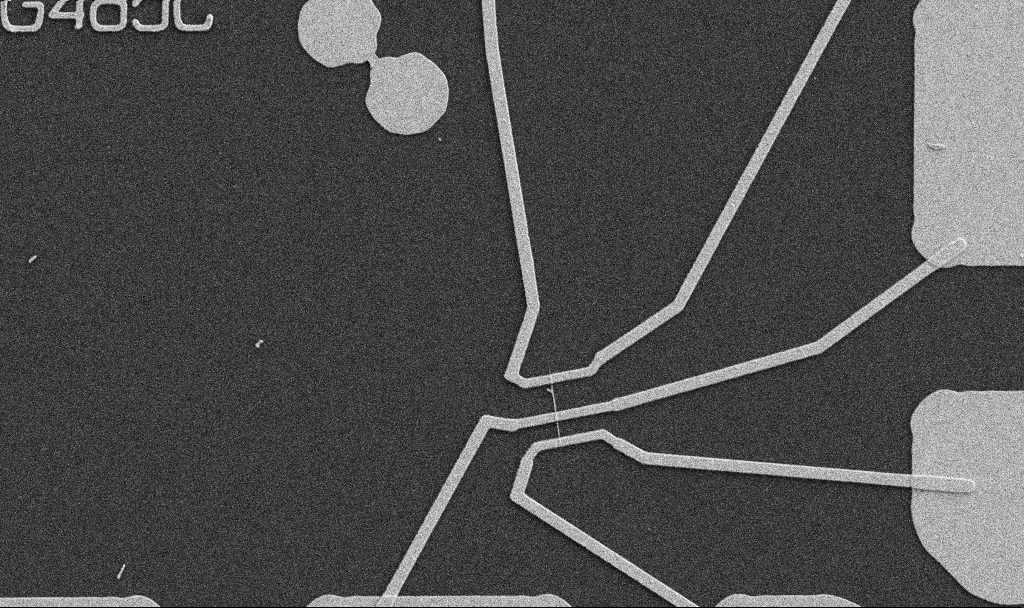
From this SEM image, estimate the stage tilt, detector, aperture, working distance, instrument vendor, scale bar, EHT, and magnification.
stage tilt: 0°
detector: SE2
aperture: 30 µm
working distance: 10.7 mm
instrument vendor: Zeiss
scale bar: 10000 nm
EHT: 5 kV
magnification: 5 K X